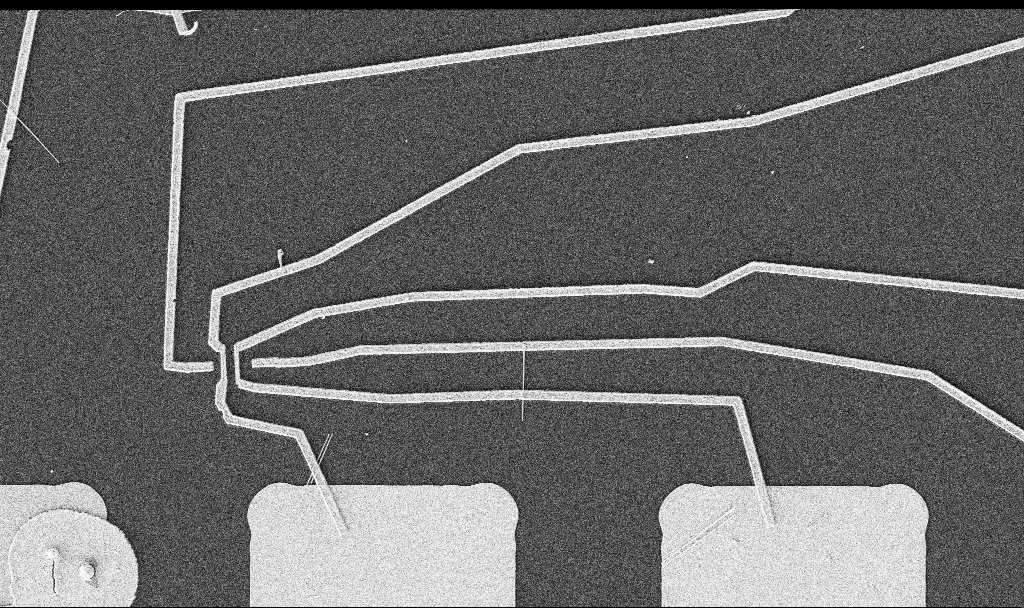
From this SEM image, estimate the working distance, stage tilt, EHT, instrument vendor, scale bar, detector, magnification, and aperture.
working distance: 10.8 mm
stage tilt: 0°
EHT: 5 kV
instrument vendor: Zeiss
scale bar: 10000 nm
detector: SE2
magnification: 5 K X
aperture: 30 µm